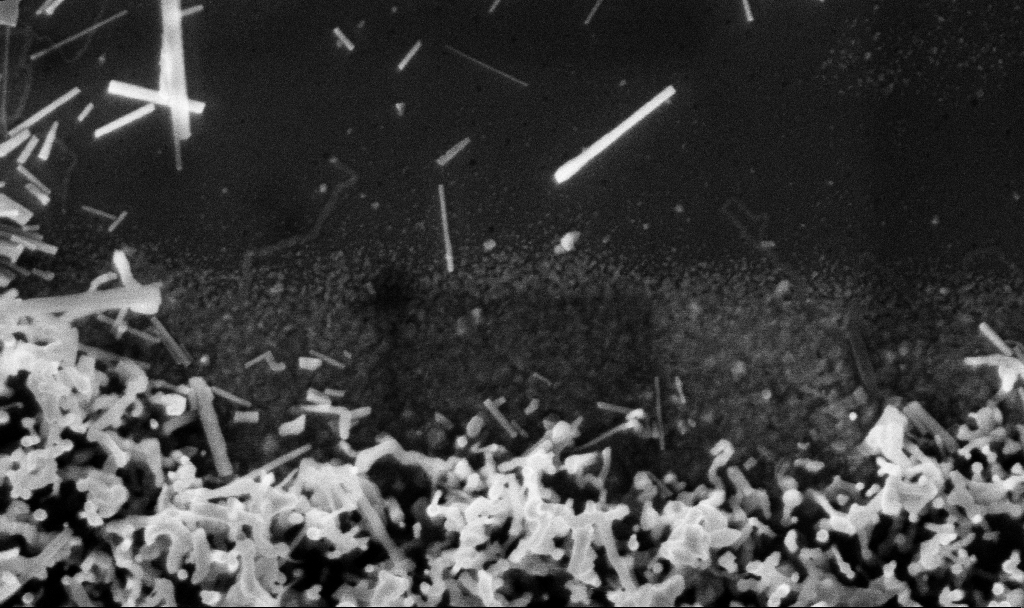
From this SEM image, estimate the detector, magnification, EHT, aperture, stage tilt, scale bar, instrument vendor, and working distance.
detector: InLens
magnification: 125.57 K X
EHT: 3 kV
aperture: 30 µm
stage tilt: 0°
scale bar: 200 nm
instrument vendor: Zeiss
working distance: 3.1 mm